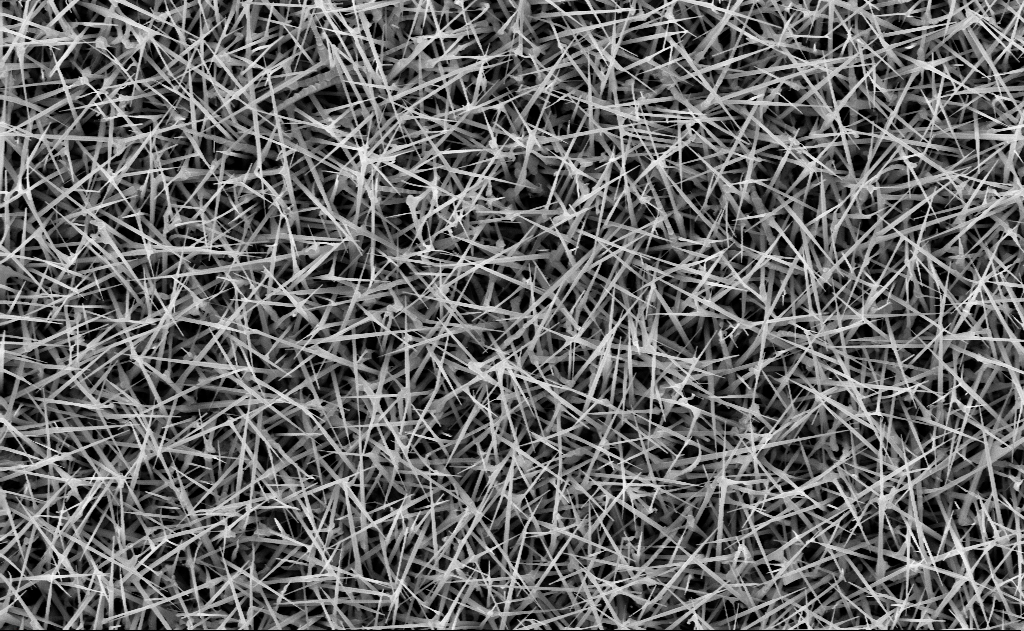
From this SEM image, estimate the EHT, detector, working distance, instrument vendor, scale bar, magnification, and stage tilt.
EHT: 10 kV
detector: InLens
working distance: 15 mm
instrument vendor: Zeiss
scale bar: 2000 nm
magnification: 20 K X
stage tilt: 0°